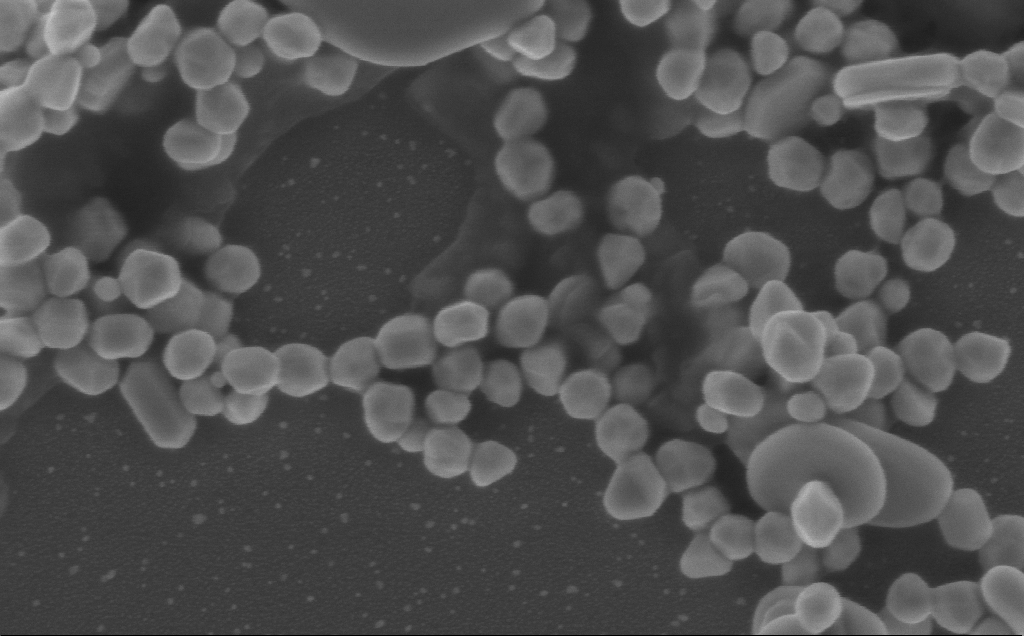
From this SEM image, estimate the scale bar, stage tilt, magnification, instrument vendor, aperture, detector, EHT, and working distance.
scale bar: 100 nm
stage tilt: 0°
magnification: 150 K X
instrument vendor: Zeiss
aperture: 30 µm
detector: InLens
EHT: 5 kV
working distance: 3 mm